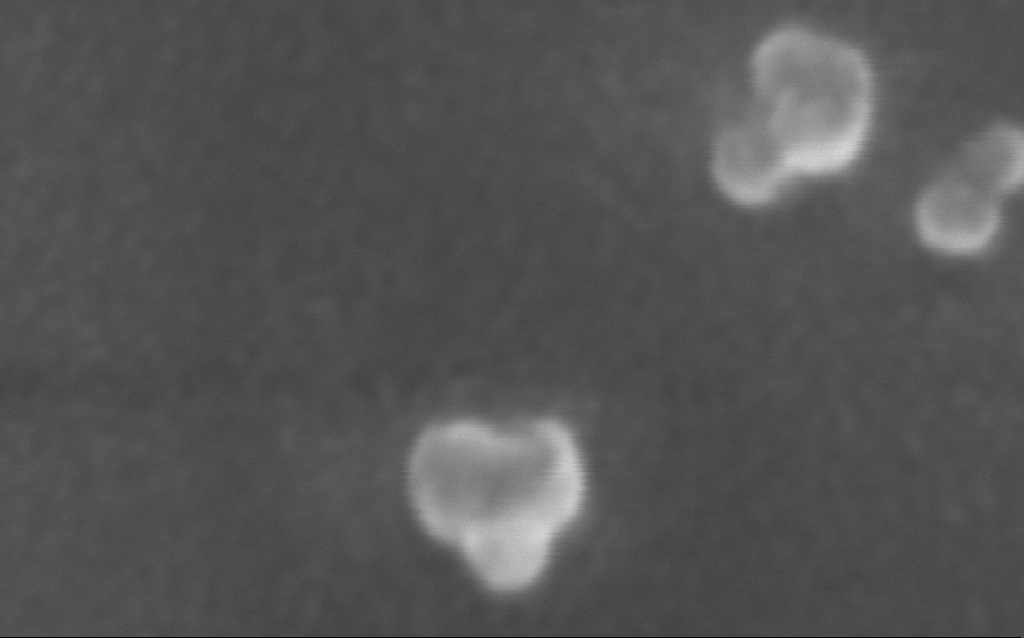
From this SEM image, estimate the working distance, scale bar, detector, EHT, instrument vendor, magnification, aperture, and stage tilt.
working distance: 5 mm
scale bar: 20 nm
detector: InLens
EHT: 10 kV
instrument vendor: Zeiss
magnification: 1905.41 K X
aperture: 30 µm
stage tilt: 0°